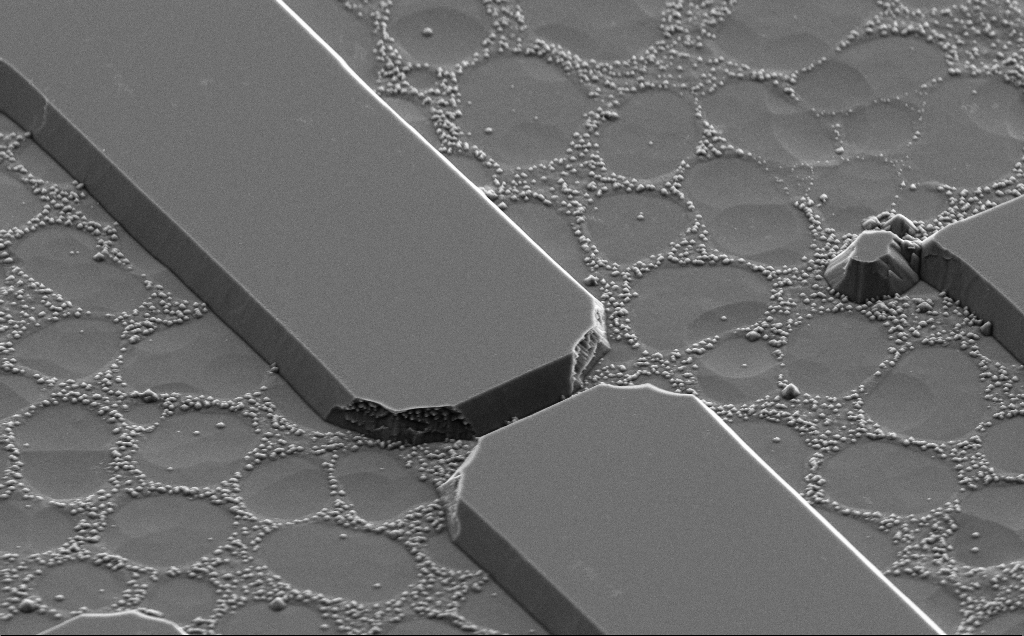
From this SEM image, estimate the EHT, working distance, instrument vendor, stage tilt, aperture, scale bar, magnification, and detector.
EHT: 5 kV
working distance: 10 mm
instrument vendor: Zeiss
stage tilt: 50°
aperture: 30 µm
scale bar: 10000 nm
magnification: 5.71 K X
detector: SE2